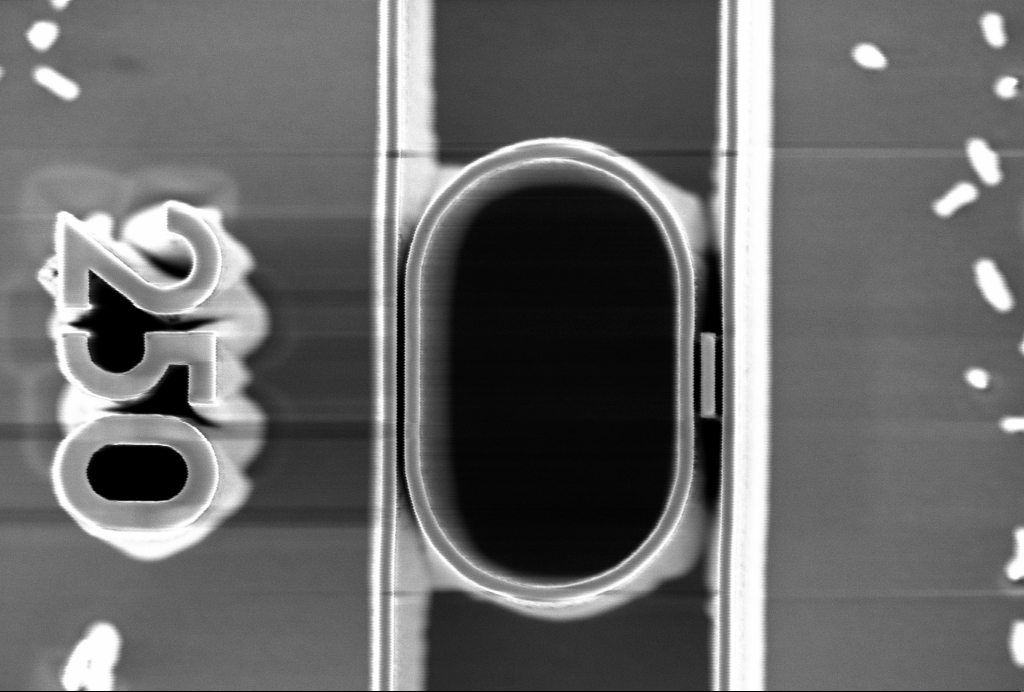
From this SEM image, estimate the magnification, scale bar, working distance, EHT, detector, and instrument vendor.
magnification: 10.7 K X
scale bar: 2000 nm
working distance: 5 mm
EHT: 5 kV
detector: InLens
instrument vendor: Zeiss